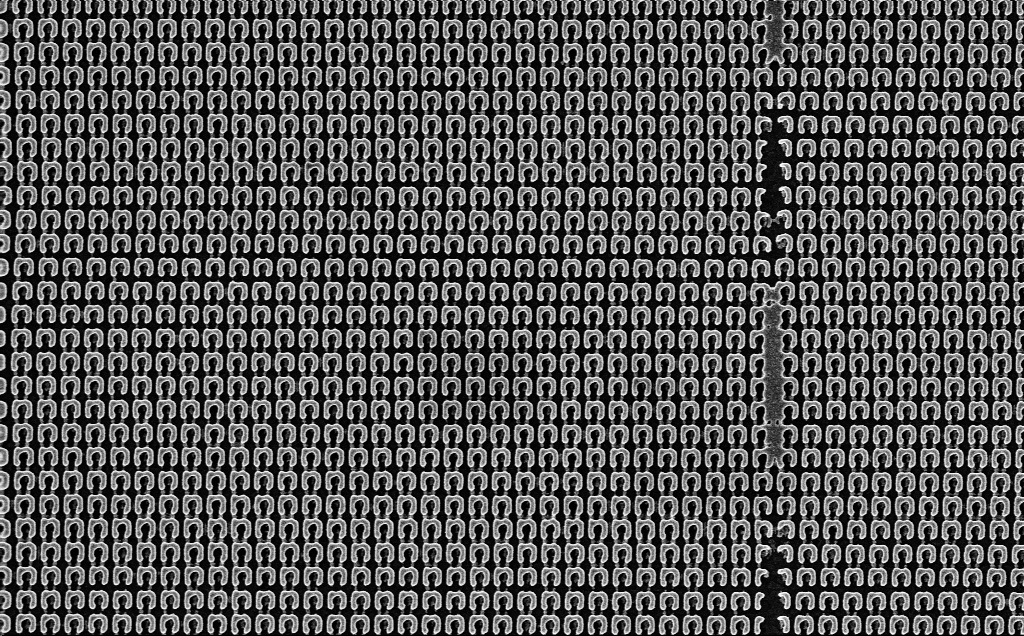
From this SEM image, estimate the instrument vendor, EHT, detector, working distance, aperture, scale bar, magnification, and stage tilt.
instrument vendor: Zeiss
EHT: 5 kV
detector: InLens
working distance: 2.5 mm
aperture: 30 µm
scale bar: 2000 nm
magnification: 18.94 K X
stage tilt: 0°